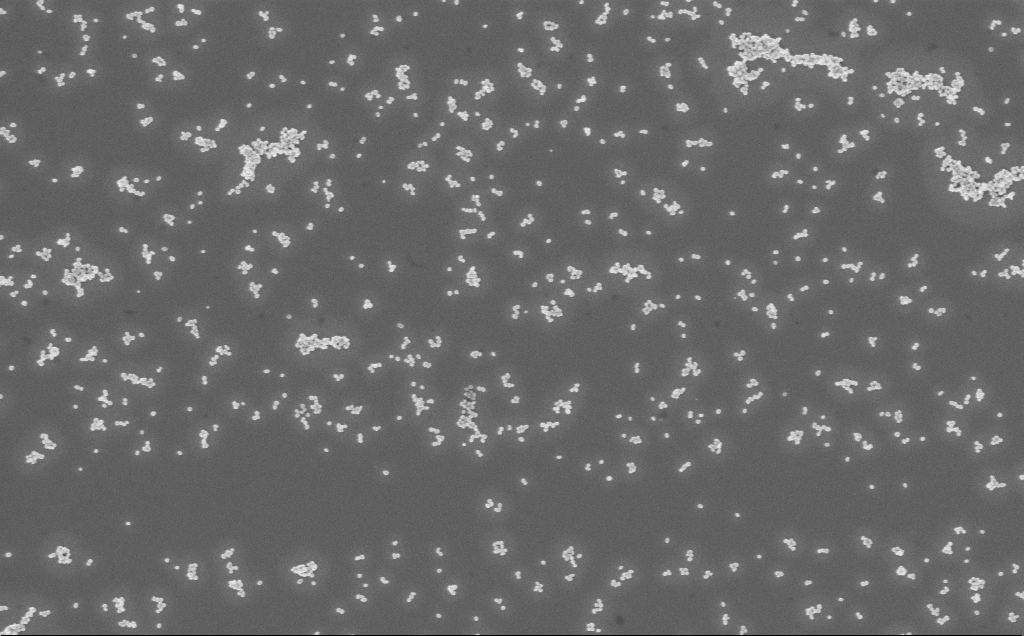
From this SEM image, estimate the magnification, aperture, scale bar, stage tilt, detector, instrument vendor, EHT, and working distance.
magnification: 40 K X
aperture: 30 µm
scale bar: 1000 nm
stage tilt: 0°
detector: InLens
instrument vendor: Zeiss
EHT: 3 kV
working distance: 5 mm